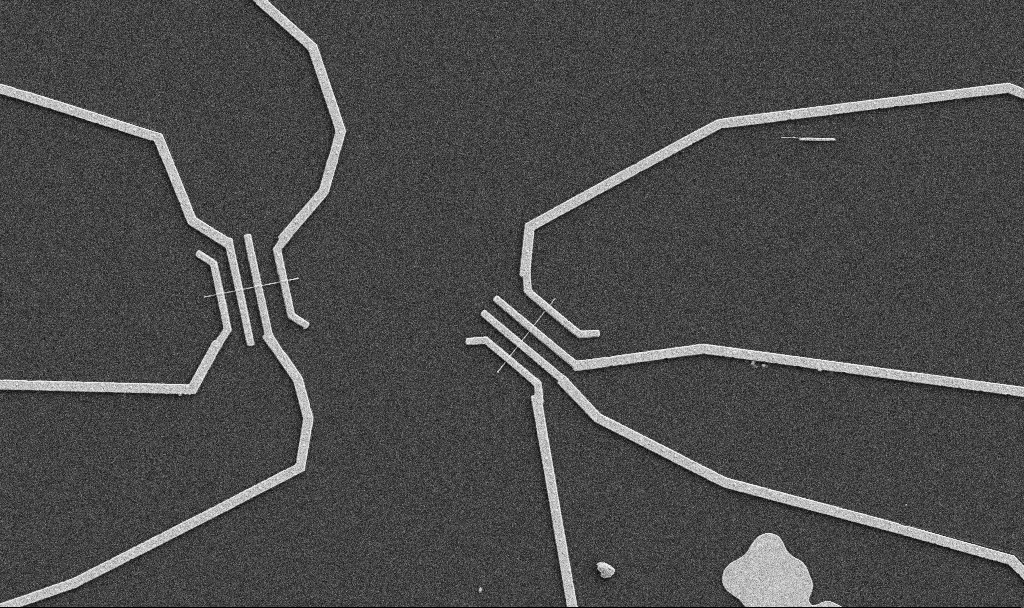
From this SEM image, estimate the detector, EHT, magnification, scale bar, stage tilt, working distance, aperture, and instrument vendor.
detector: SE2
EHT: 5 kV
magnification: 5 K X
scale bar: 10000 nm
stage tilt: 0°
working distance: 10.7 mm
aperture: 30 µm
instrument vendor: Zeiss